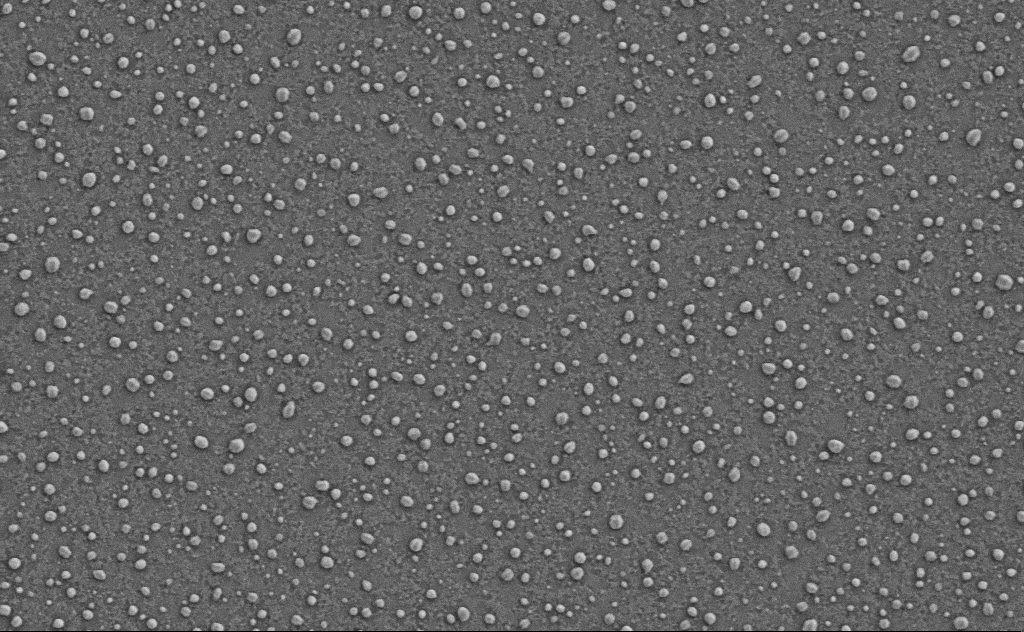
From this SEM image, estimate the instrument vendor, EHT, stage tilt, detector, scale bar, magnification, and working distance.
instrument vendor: Zeiss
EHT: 3 kV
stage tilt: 0°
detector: SE2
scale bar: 1000 nm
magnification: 40 K X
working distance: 4 mm